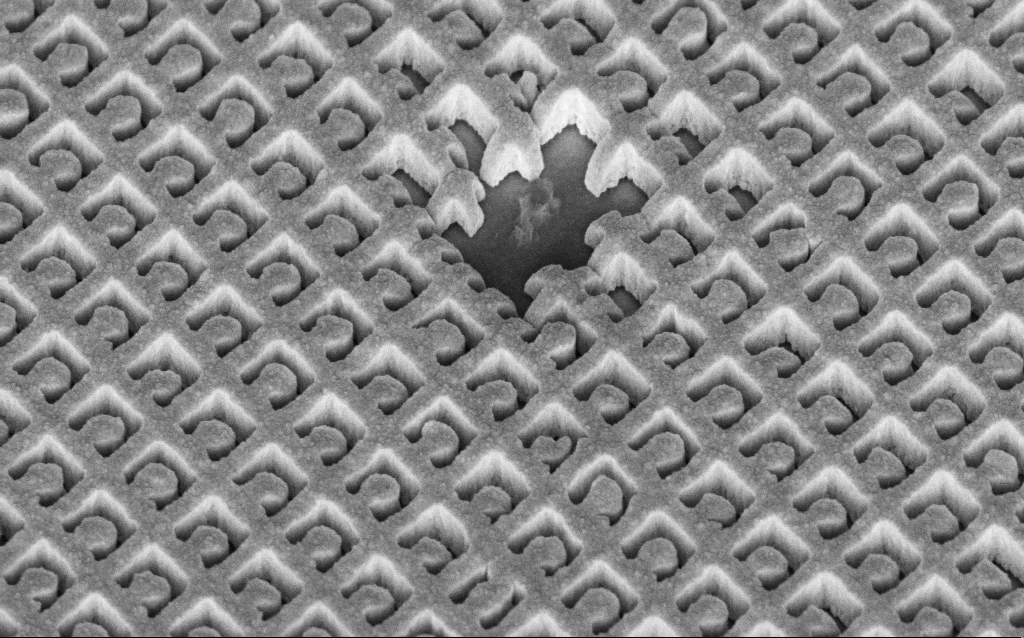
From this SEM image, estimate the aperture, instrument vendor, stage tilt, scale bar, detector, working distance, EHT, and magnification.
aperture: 30 µm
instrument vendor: Zeiss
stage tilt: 45°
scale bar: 1000 nm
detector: InLens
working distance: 7.3 mm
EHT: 5 kV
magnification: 63.26 K X